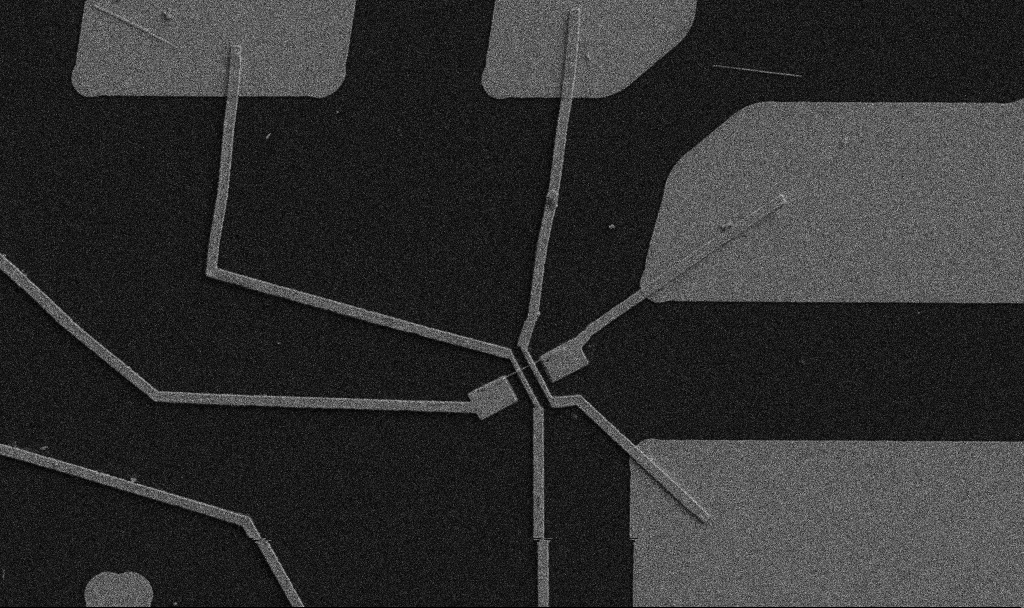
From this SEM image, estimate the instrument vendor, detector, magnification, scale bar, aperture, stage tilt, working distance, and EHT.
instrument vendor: Zeiss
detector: SE2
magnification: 5 K X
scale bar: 10000 nm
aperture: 30 µm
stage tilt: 0°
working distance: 10.7 mm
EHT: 5 kV